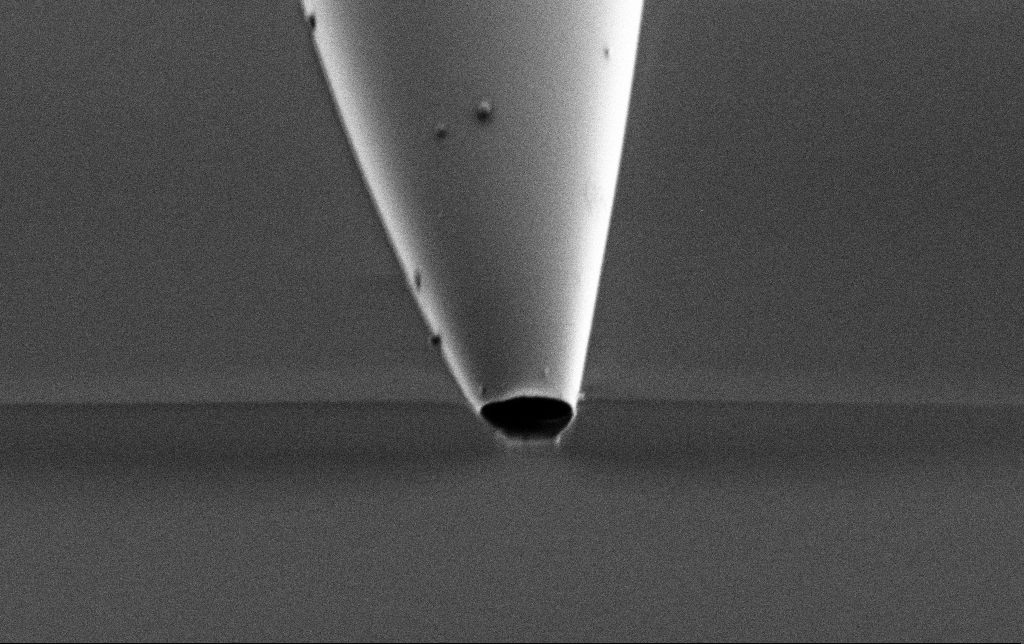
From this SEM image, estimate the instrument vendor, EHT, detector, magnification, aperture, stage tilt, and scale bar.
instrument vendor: Zeiss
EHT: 1 kV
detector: SE2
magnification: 25 K X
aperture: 30 µm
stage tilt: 45°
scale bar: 2000 nm